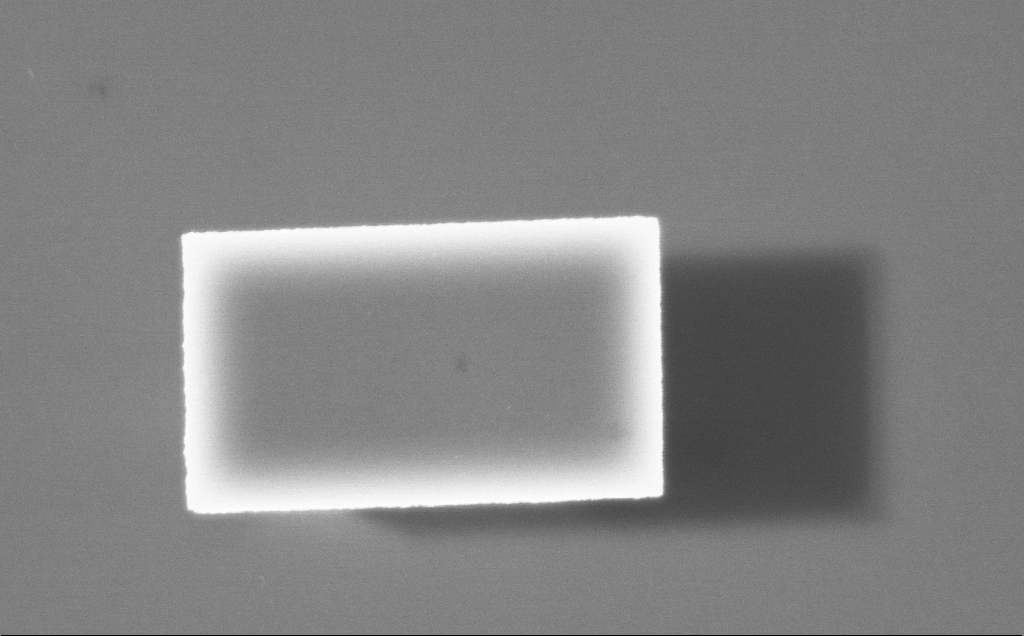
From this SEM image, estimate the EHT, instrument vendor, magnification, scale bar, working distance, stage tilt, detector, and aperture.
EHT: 7.5 kV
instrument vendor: Zeiss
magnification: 35.97 K X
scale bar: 1000 nm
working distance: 4 mm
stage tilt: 0°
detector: InLens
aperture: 30 µm